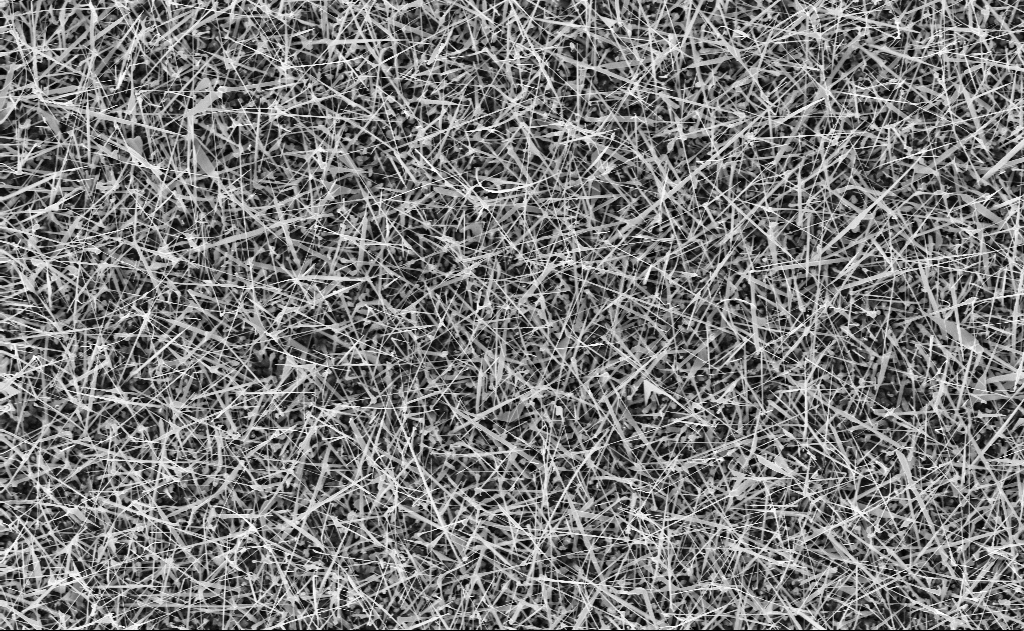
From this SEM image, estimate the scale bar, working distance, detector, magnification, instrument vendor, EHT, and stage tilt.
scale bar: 2000 nm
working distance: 10 mm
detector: InLens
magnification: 10 K X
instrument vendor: Zeiss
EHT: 10 kV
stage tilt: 0°